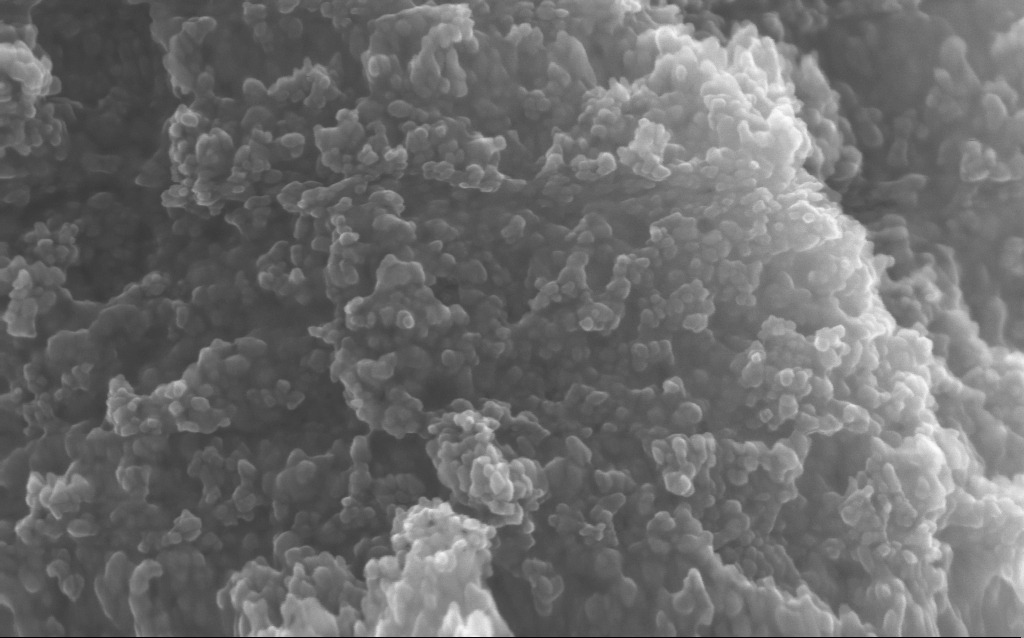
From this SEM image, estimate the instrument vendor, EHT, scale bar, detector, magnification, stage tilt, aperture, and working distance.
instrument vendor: Zeiss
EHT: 10 kV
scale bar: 100 nm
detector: InLens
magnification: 204.13 K X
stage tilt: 0°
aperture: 30 µm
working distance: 2.7 mm